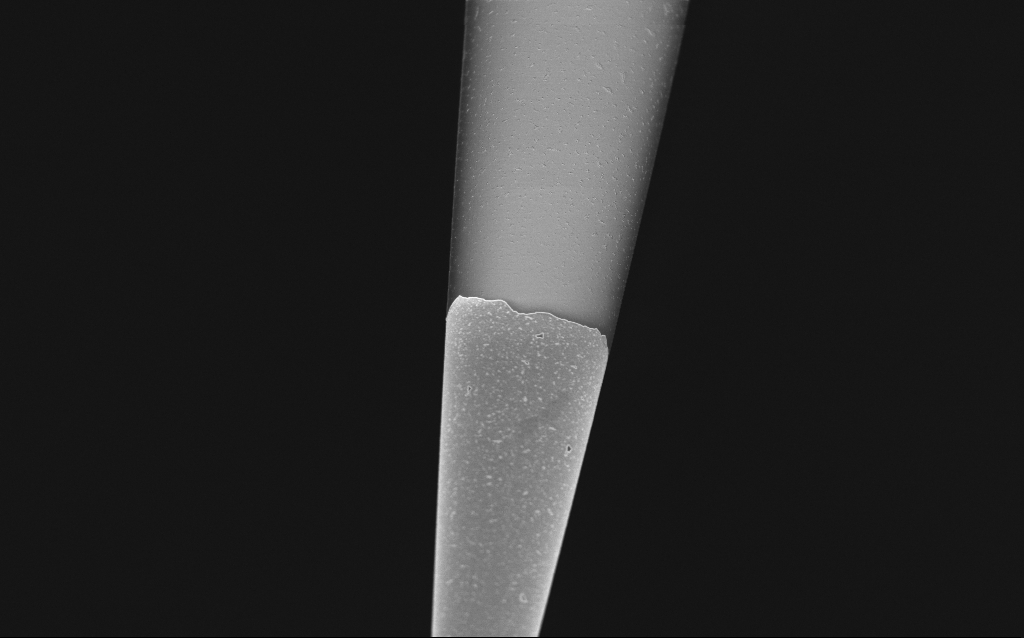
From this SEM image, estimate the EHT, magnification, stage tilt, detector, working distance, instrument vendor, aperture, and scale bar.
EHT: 1 kV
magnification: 5 K X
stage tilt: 45°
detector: InLens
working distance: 6 mm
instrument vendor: Zeiss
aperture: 30 µm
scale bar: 10000 nm